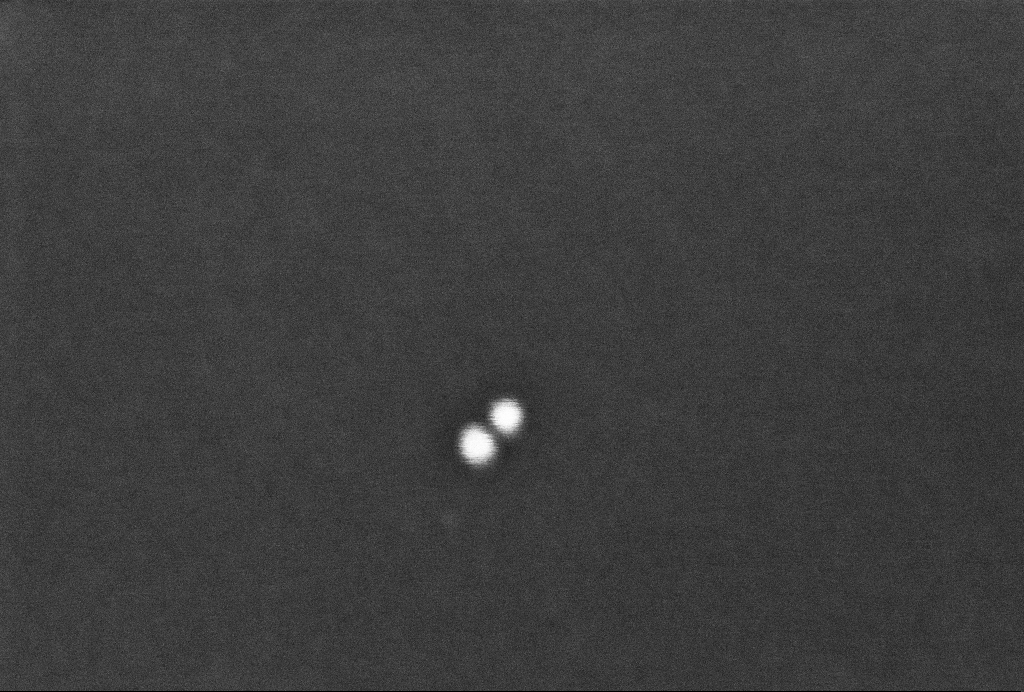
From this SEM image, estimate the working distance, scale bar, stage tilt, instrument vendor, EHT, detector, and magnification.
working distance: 3.3 mm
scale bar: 100 nm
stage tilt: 0°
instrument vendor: Zeiss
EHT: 2 kV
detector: InLens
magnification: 401.53 K X